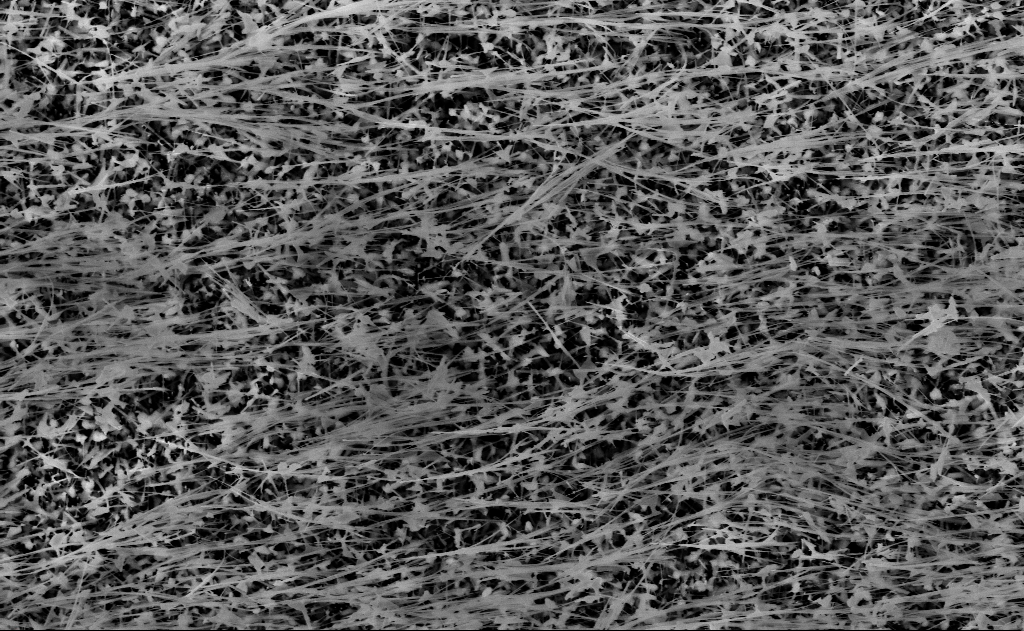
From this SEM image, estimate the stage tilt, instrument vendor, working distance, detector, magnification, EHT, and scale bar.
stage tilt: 0°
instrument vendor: Zeiss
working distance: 15 mm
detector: InLens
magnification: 20 K X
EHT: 10 kV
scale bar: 2000 nm